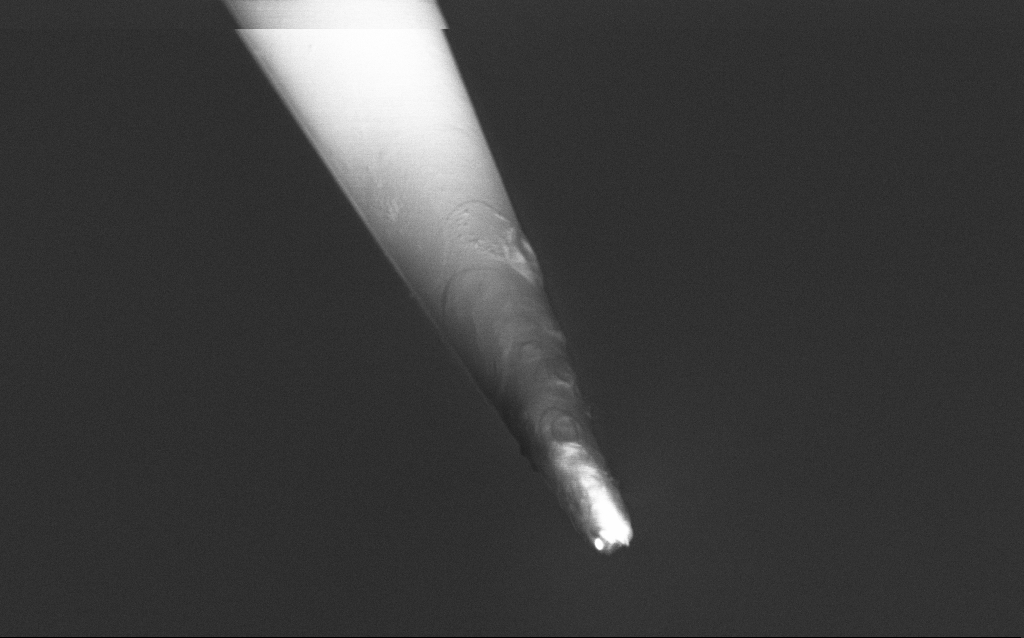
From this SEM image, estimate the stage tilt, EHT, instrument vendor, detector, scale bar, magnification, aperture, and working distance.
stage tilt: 45°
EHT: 1 kV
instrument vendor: Zeiss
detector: InLens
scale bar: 1000 nm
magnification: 25 K X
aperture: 30 µm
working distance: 6 mm